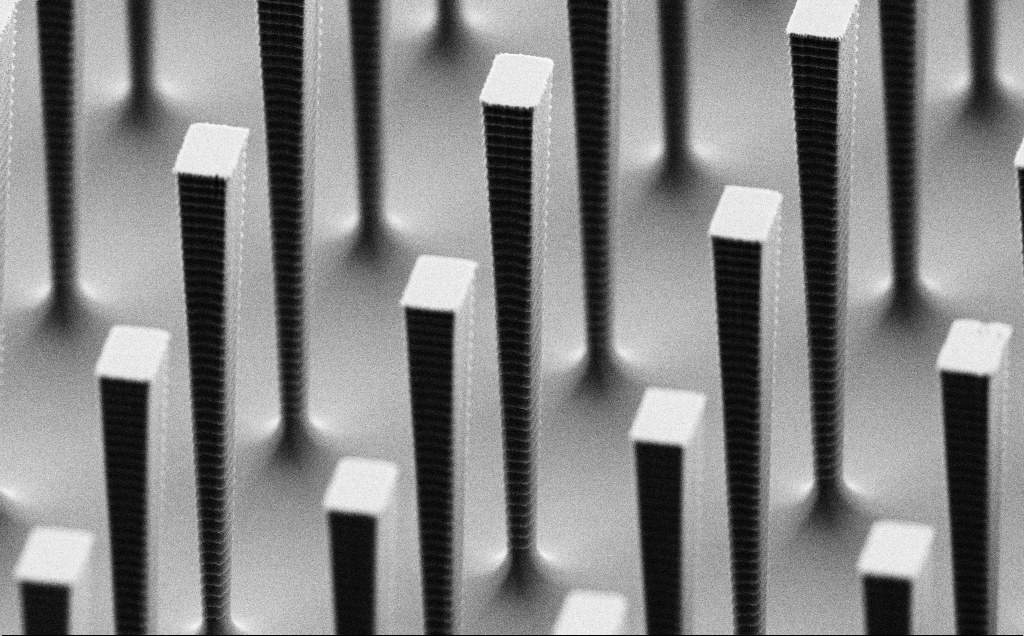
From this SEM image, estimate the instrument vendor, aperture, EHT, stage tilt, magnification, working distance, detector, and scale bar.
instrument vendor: Zeiss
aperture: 30 µm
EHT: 3 kV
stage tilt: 60°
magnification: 11.1 K X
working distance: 5.4 mm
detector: SE2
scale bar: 2000 nm